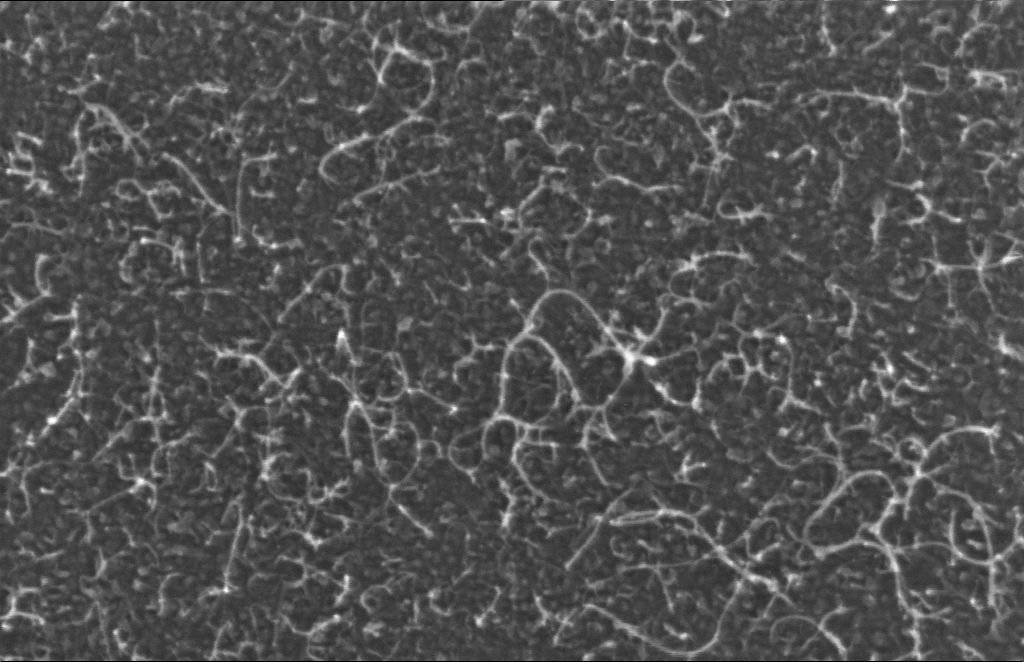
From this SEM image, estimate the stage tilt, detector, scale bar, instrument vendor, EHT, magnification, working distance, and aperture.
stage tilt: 0°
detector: InLens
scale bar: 100 nm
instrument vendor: Zeiss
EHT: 5 kV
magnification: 338.3 K X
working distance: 4 mm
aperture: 30 µm